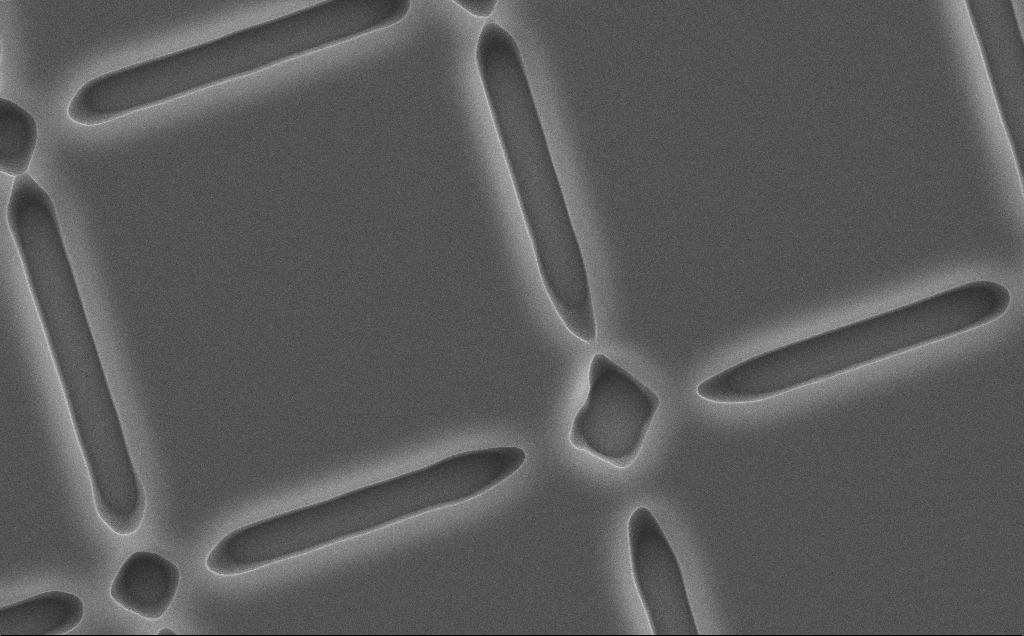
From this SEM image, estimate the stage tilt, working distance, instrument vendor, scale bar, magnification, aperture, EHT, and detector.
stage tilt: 0°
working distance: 12 mm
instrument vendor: Zeiss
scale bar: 2000 nm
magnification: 8.75 K X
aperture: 30 µm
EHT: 10 kV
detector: SE2